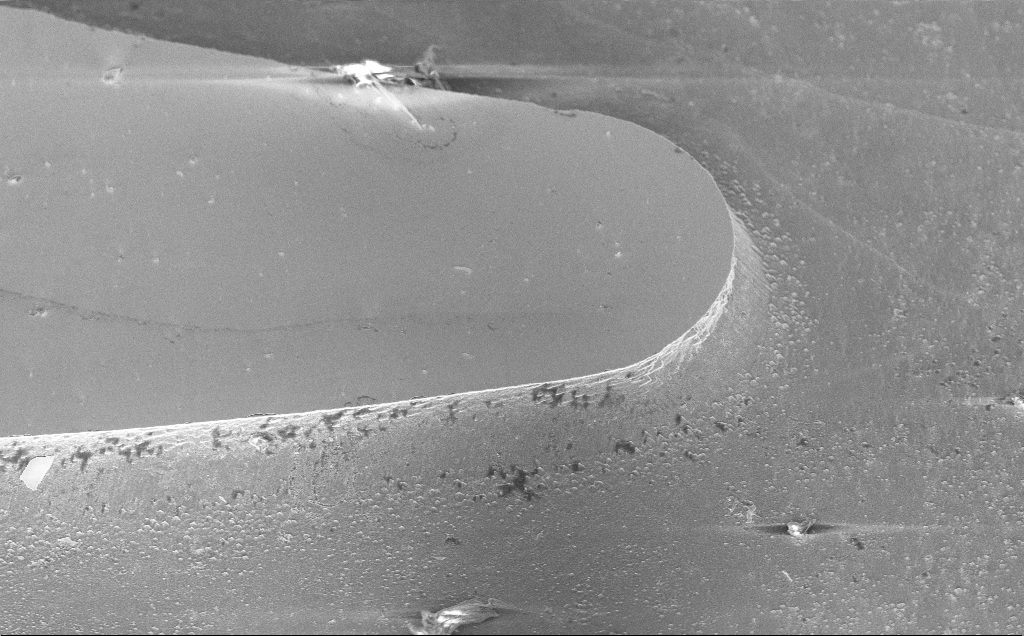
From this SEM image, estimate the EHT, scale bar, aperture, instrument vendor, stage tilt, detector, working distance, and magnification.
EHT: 5 kV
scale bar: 10000 nm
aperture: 30 µm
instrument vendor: Zeiss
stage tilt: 45°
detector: InLens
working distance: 10 mm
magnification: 2.21 K X